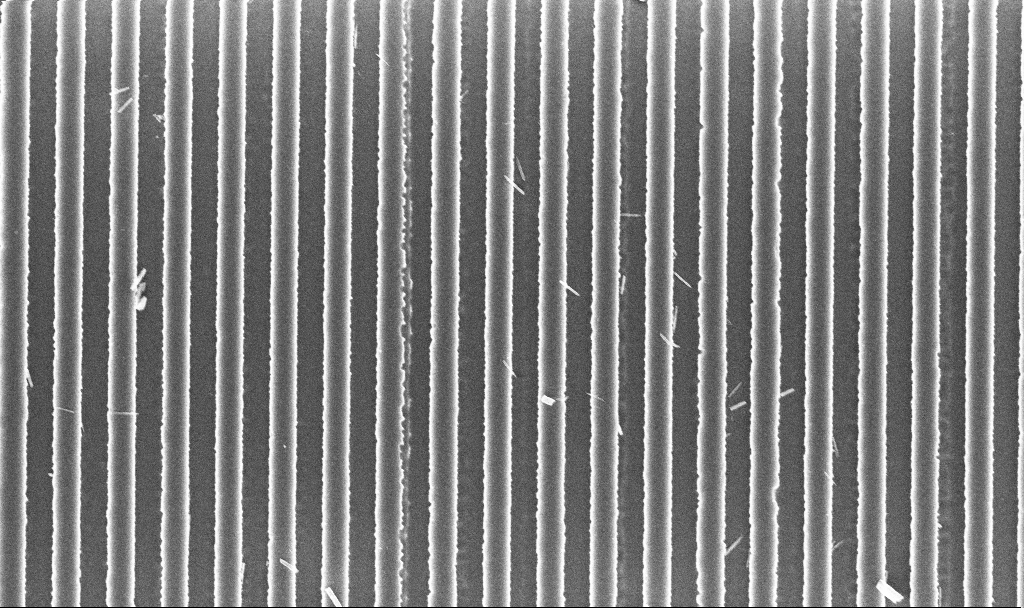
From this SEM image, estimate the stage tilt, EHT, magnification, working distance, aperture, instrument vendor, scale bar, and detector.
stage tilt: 0°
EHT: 3 kV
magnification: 30.21 K X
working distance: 3 mm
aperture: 30 µm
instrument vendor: Zeiss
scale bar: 2000 nm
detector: InLens